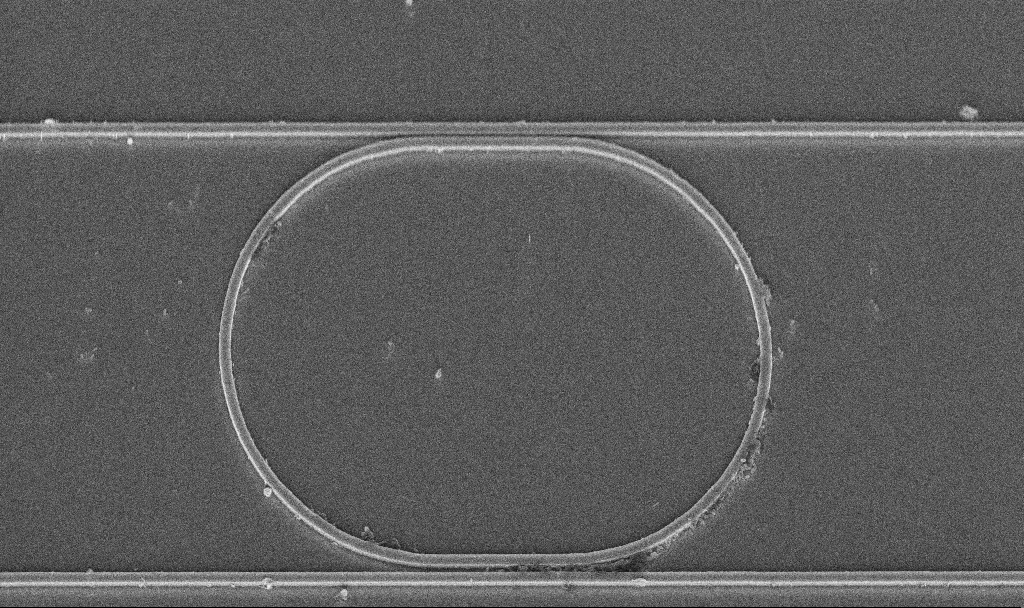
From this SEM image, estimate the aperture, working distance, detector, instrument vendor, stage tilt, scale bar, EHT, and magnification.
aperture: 30 µm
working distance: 9.4 mm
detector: InLens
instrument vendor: Zeiss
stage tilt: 45°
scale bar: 2000 nm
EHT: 5 kV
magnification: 7.8 K X